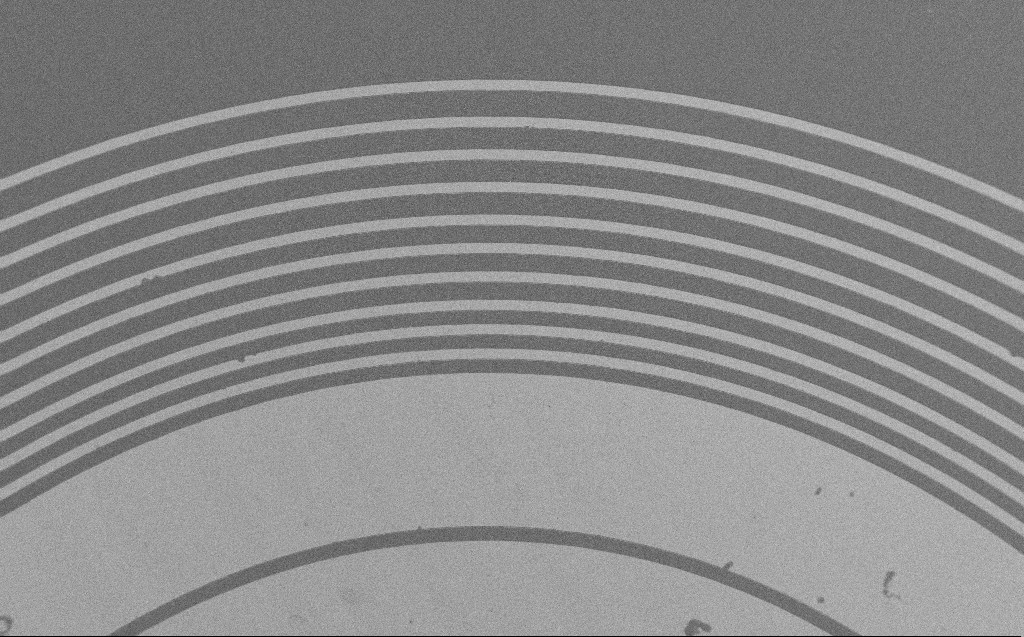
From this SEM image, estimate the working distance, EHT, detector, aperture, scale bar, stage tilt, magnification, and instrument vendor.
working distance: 4 mm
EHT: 1.2 kV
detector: SE2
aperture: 30 µm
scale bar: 200000 nm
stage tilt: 0°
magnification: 0.303 K X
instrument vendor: Zeiss